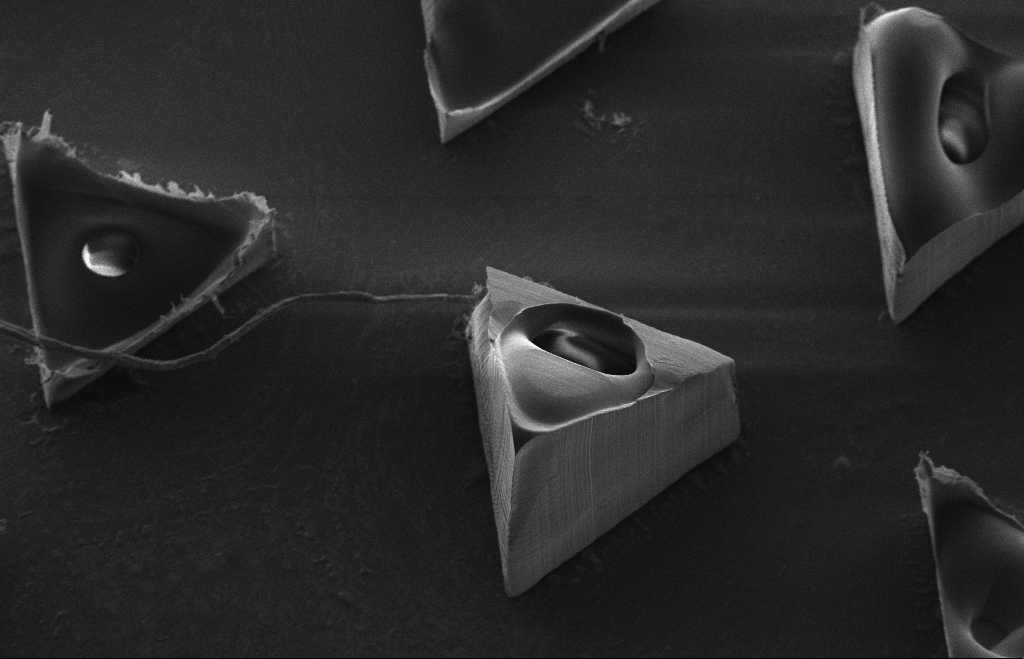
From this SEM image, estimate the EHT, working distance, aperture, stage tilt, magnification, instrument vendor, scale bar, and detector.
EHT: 5 kV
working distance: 14 mm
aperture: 30 µm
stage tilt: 17.3°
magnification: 0.381 K X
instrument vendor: Zeiss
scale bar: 100000 nm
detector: SE2